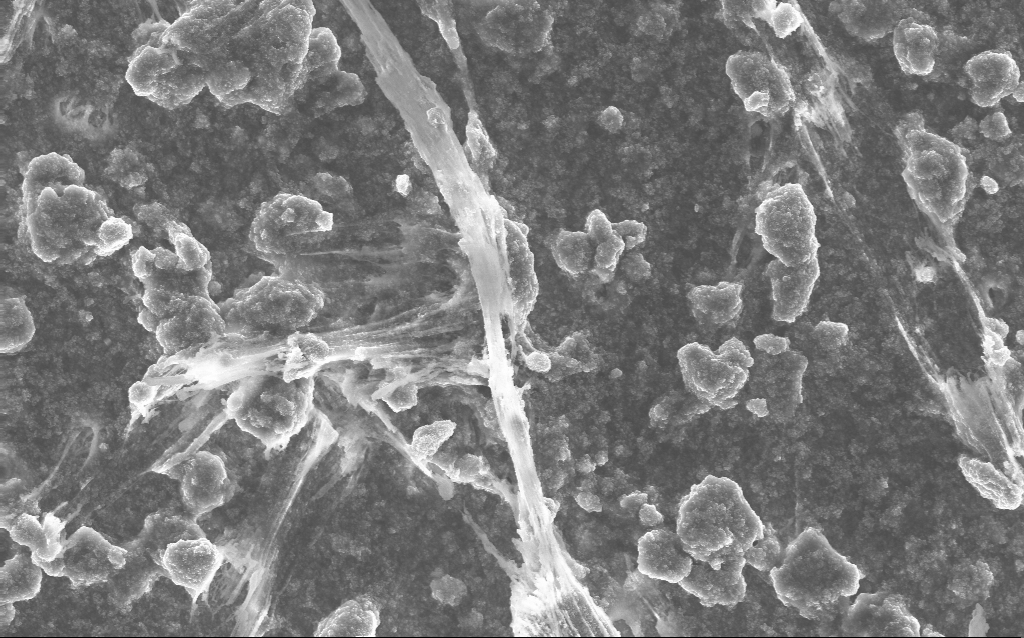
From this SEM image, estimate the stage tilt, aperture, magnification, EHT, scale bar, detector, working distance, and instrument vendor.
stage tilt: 0°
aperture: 30 µm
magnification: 5.83 K X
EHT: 10 kV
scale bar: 10000 nm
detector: InLens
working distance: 2.8 mm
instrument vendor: Zeiss